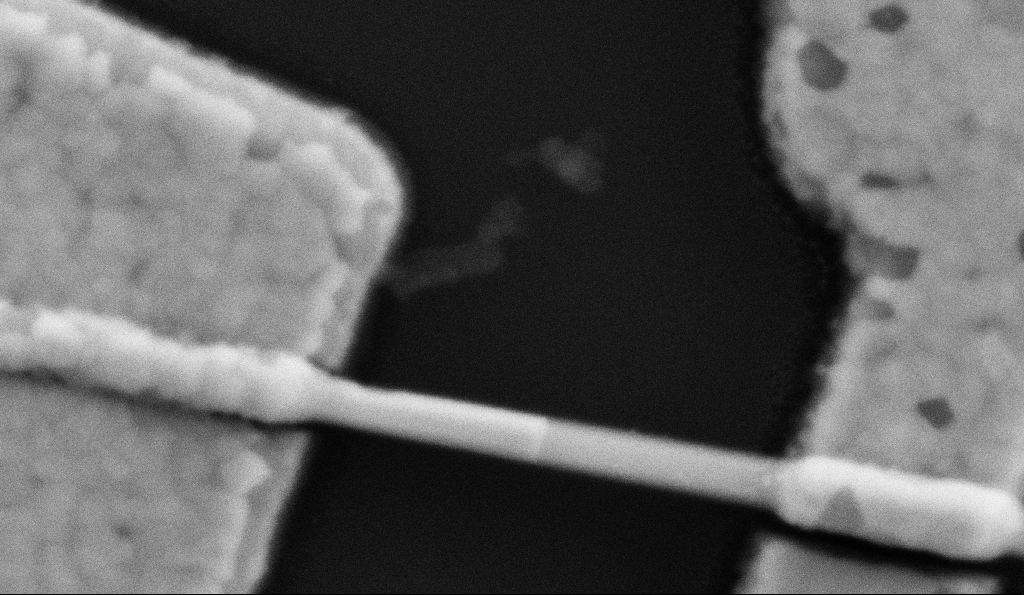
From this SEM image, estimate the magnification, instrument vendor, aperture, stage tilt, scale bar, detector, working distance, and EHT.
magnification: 200 K X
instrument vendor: Zeiss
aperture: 30 µm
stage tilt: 0°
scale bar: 200 nm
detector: SE2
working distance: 8.5 mm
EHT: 5 kV